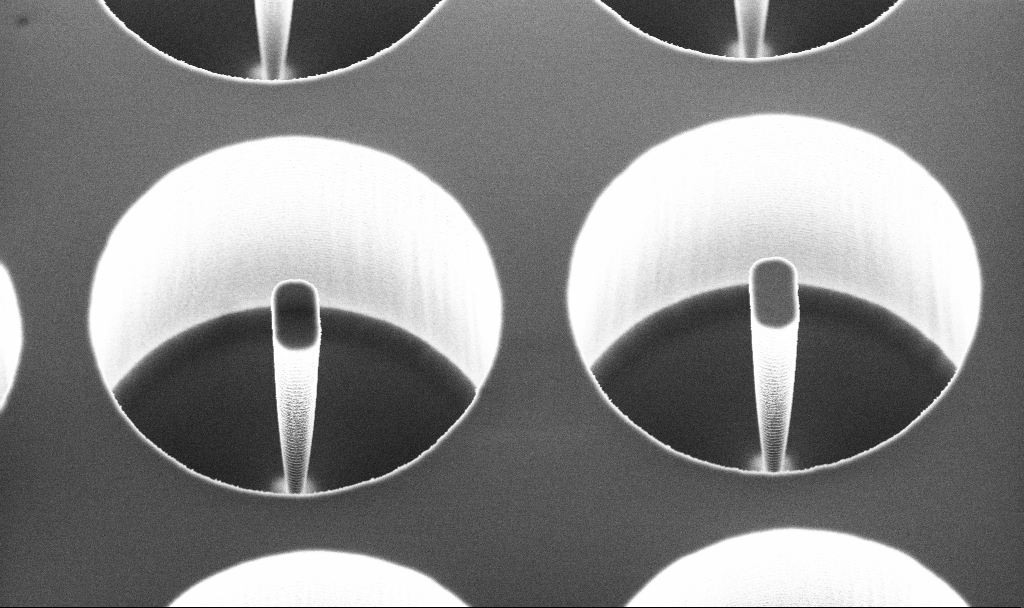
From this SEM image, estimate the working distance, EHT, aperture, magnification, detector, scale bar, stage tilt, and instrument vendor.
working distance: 6.8 mm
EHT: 5 kV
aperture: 30 µm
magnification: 7.69 K X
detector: InLens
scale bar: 2000 nm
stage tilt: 29.2°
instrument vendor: Zeiss